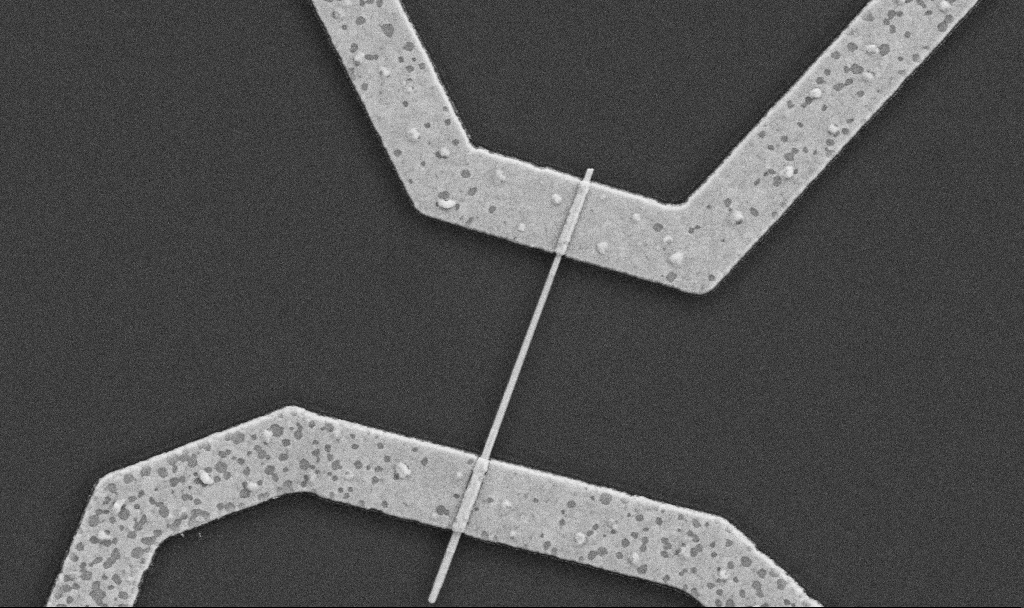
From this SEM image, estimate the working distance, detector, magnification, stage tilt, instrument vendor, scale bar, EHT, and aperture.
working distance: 8.7 mm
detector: SE2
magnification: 30 K X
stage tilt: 0°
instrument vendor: Zeiss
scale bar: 1000 nm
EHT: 5 kV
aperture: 30 µm